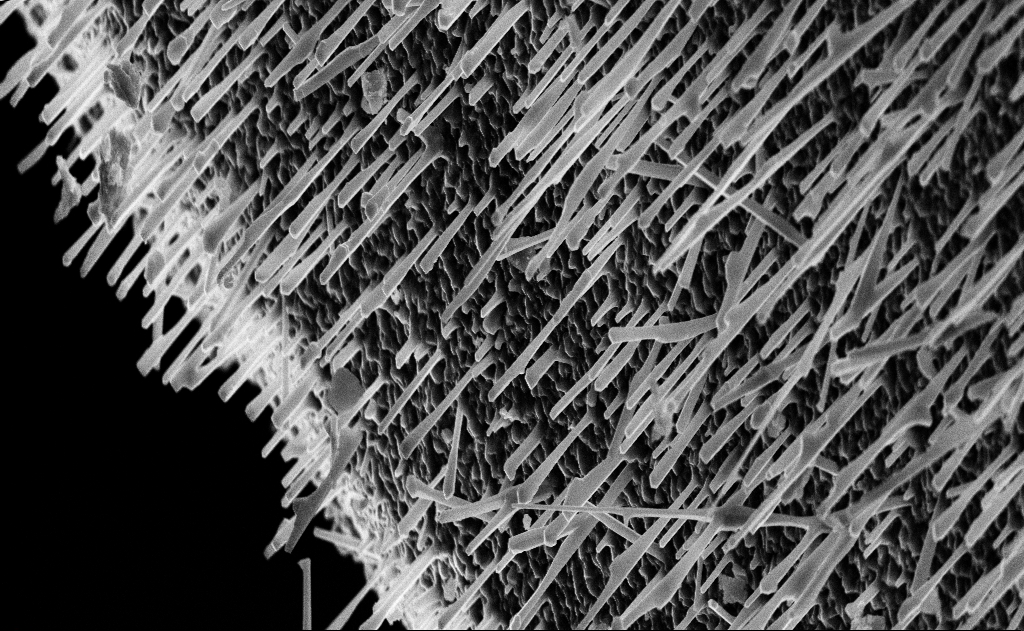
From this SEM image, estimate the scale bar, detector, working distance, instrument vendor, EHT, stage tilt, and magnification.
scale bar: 2000 nm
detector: InLens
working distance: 15 mm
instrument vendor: Zeiss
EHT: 10 kV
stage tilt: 0°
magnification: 10 K X